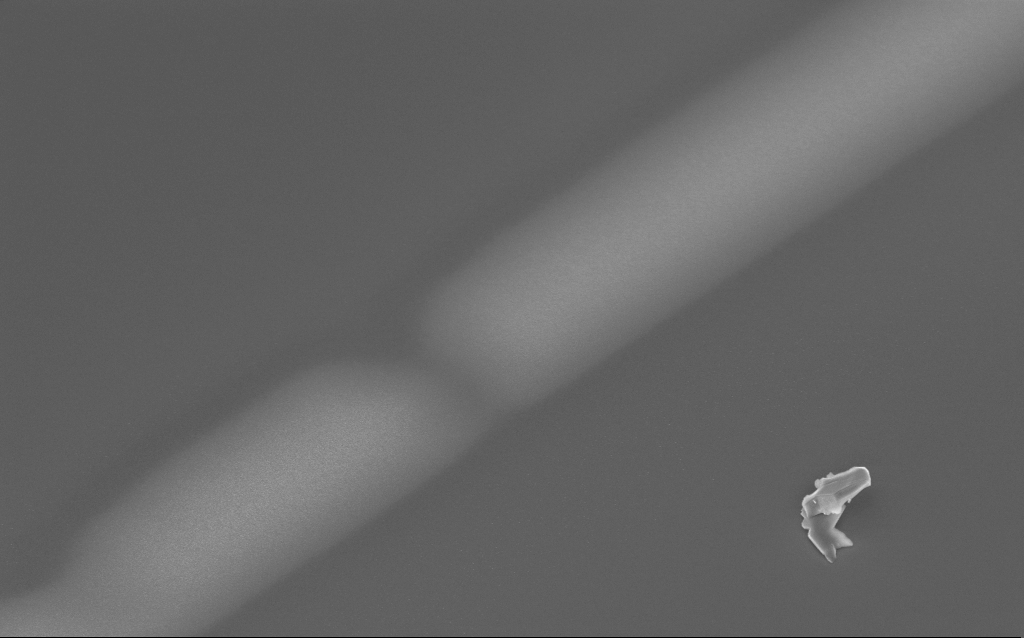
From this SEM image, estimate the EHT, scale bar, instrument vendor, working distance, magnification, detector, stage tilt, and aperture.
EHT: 3 kV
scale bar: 2000 nm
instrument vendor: Zeiss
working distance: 2 mm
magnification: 15.6 K X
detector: InLens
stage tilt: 30°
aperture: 30 µm